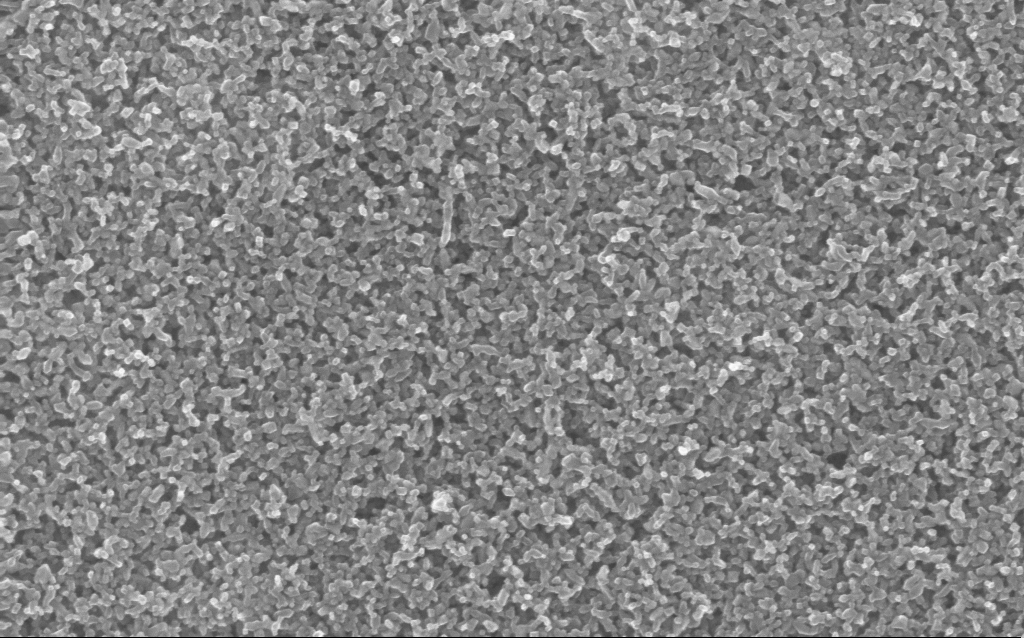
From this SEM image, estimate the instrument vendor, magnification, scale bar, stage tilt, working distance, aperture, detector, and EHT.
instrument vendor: Zeiss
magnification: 130 K X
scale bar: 100 nm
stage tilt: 0°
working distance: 5 mm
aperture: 30 µm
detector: InLens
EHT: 10 kV